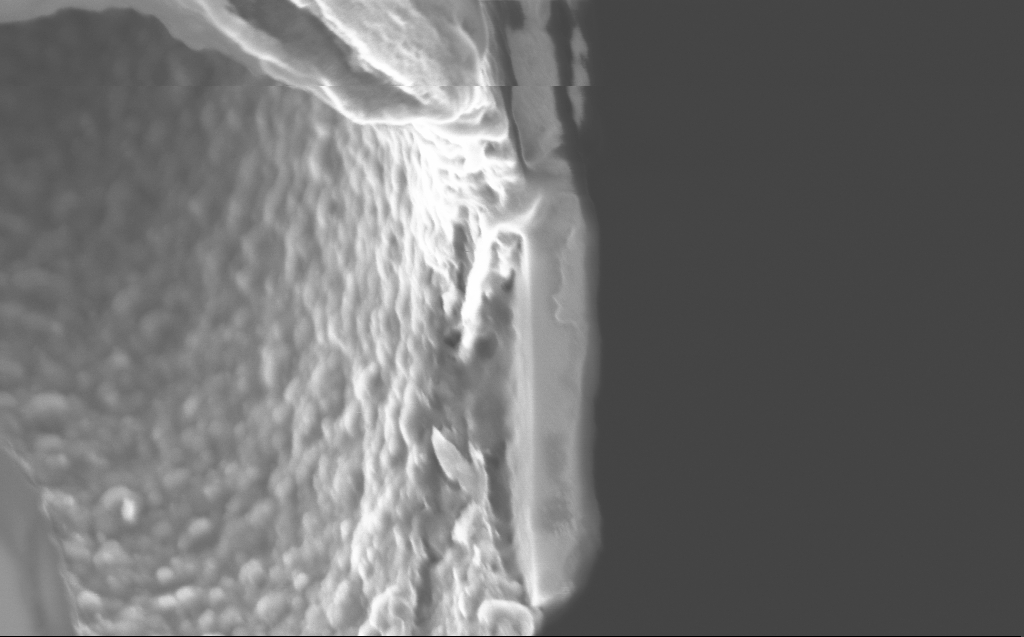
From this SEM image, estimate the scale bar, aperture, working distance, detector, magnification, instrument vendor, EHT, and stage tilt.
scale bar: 200 nm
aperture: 30 µm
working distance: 2 mm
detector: InLens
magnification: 104.64 K X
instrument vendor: Zeiss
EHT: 2 kV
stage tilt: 45°